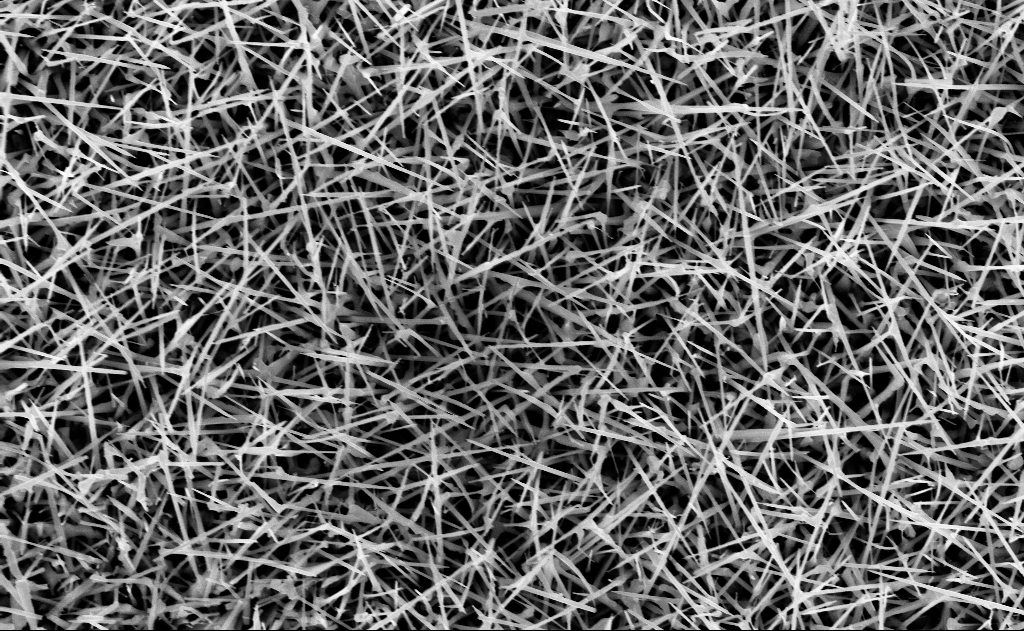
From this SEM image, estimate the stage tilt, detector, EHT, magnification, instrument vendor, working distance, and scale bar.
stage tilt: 0°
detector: InLens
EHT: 10 kV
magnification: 20 K X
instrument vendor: Zeiss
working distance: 13 mm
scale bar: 2000 nm